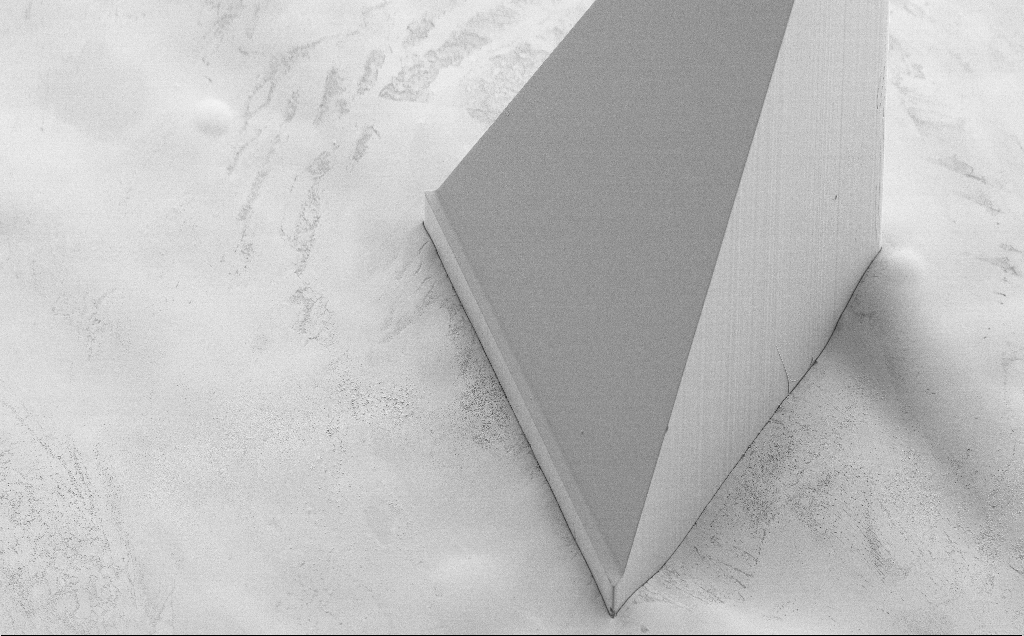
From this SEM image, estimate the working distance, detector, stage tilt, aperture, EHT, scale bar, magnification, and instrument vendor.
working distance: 9 mm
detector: SE2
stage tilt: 40°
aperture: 30 µm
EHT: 5 kV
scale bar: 100000 nm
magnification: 0.274 K X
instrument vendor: Zeiss